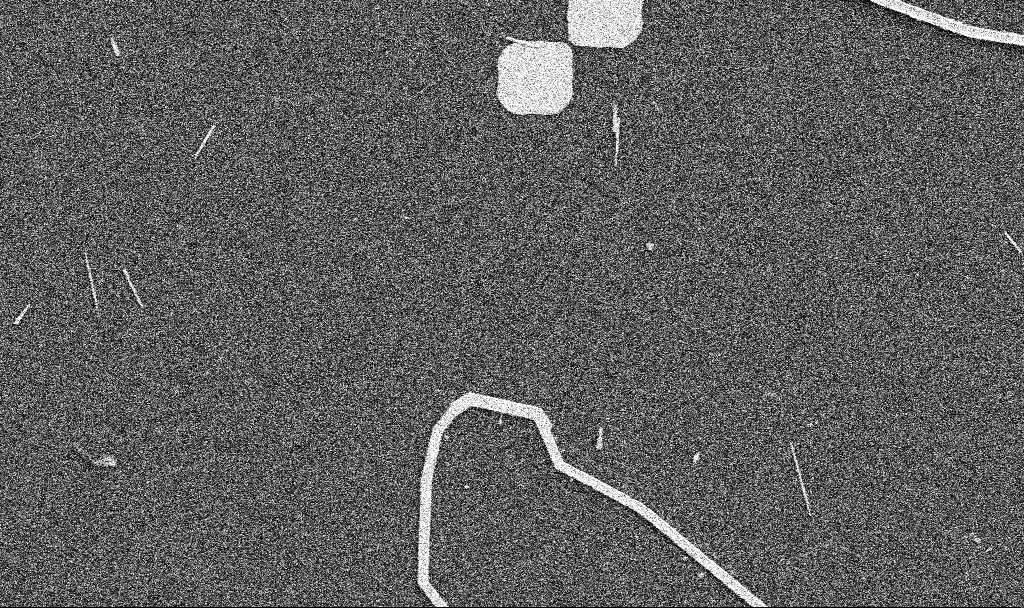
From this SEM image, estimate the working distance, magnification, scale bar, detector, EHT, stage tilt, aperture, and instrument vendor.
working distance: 10.7 mm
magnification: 5 K X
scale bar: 10000 nm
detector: SE2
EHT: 5 kV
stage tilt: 0°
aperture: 30 µm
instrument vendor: Zeiss